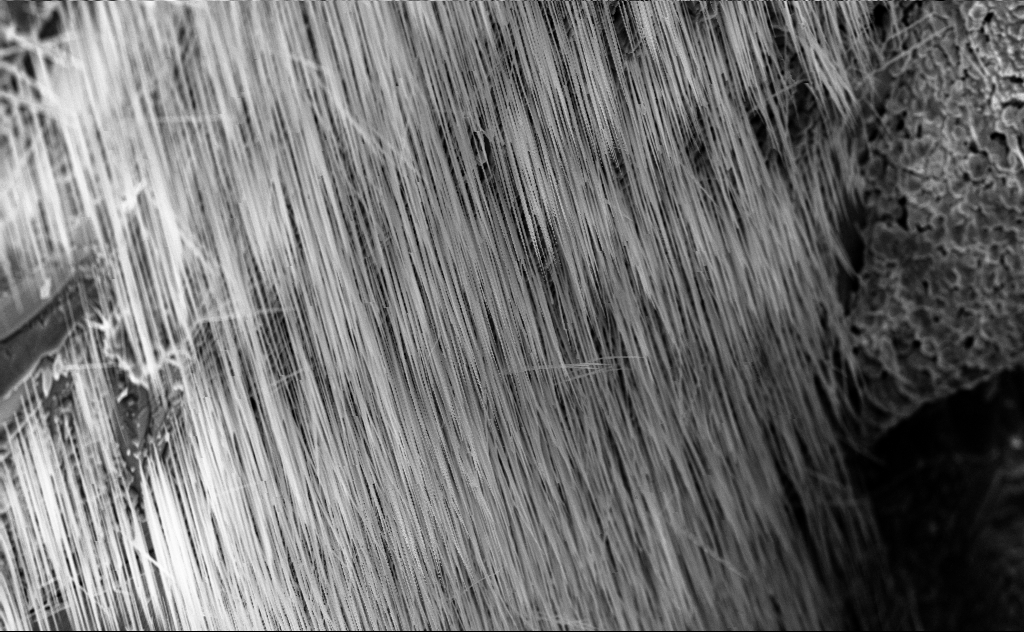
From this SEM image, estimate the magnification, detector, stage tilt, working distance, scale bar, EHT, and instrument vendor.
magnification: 5.61 K X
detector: InLens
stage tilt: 45°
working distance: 6 mm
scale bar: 10000 nm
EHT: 10 kV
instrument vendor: Zeiss